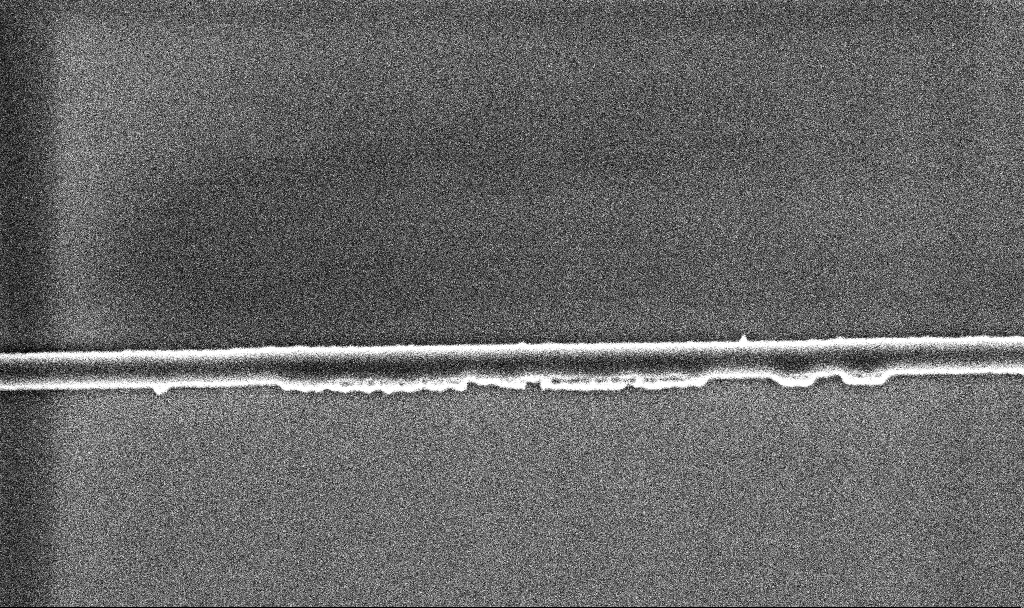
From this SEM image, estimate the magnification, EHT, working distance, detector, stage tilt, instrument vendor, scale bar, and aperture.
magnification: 26.25 K X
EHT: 5 kV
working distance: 5.2 mm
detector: InLens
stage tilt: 0°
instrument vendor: Zeiss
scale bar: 2000 nm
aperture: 30 µm